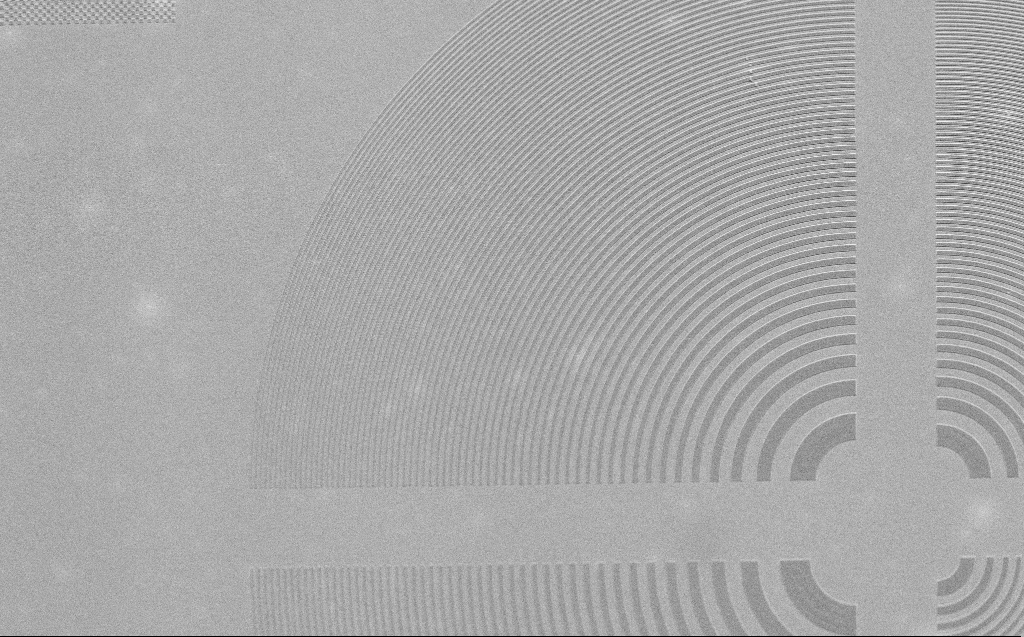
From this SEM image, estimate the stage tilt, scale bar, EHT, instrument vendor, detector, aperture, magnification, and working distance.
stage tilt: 30°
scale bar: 20000 nm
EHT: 2.5 kV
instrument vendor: Zeiss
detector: SE2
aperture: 30 µm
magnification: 2.97 K X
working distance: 5 mm